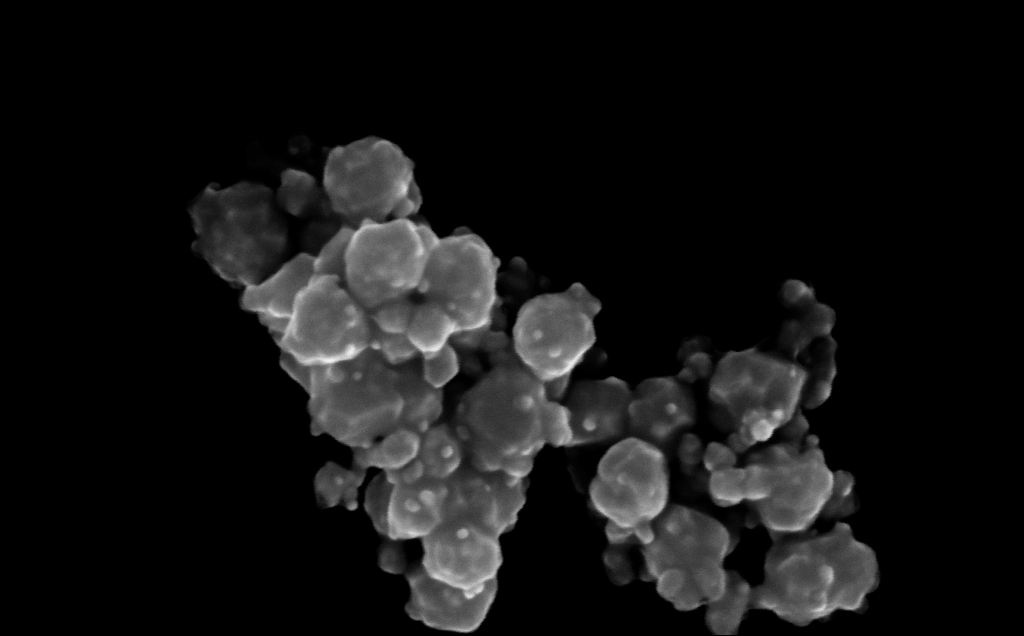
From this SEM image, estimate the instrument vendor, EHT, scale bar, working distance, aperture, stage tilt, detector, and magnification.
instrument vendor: Zeiss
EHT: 10 kV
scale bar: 200 nm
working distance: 4 mm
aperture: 30 µm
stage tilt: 0°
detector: InLens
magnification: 312.25 K X